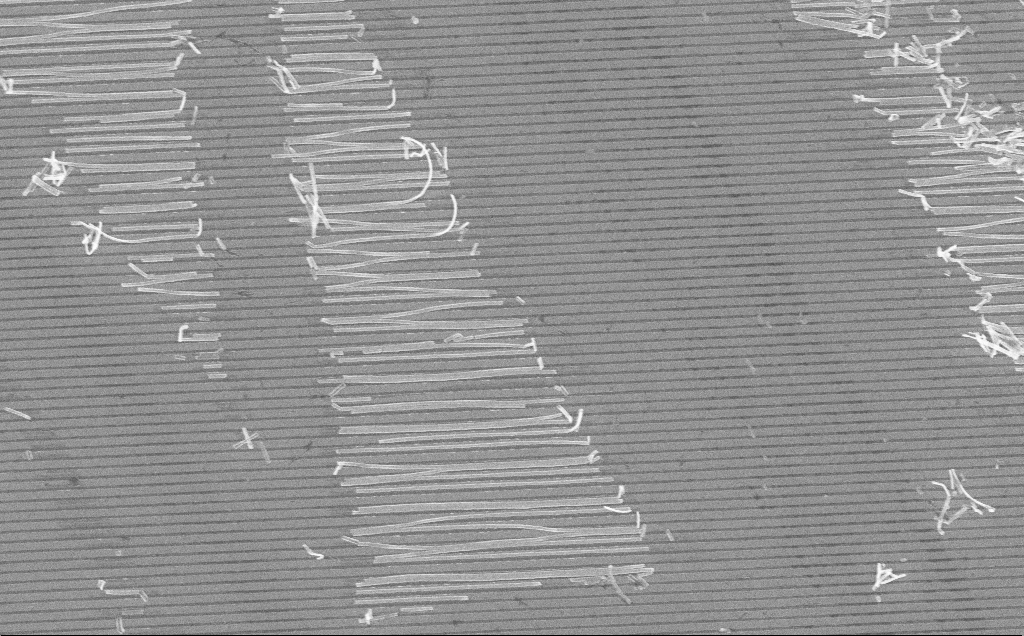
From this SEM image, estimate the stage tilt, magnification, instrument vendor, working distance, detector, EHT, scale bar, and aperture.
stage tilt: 0°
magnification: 14.93 K X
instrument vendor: Zeiss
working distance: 7 mm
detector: InLens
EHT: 10 kV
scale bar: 2000 nm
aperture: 30 µm